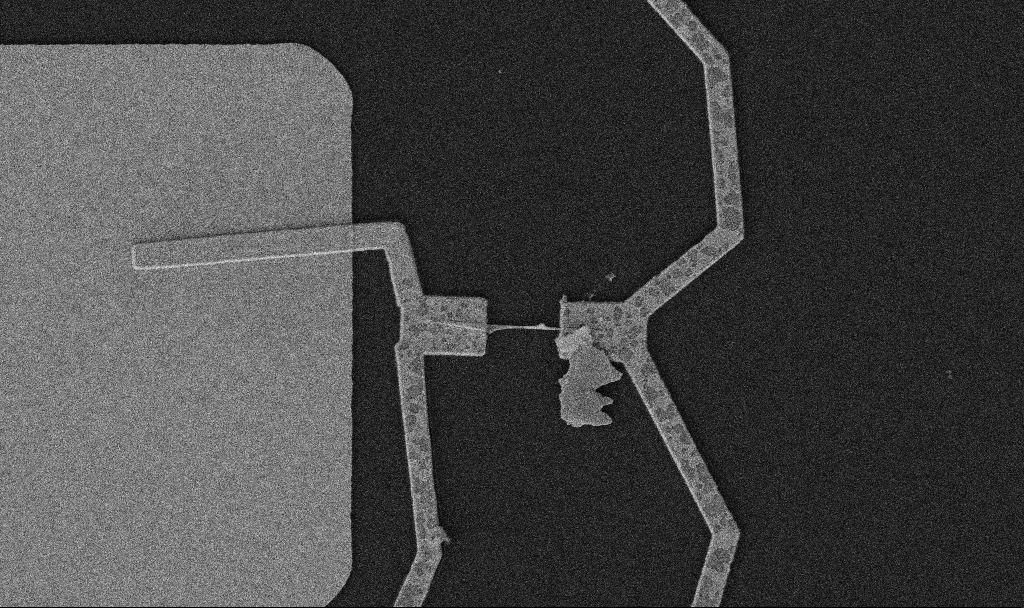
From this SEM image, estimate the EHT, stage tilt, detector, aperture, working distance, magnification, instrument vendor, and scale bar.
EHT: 5 kV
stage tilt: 0°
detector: SE2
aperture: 30 µm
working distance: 10.7 mm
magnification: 10 K X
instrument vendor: Zeiss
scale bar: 2000 nm